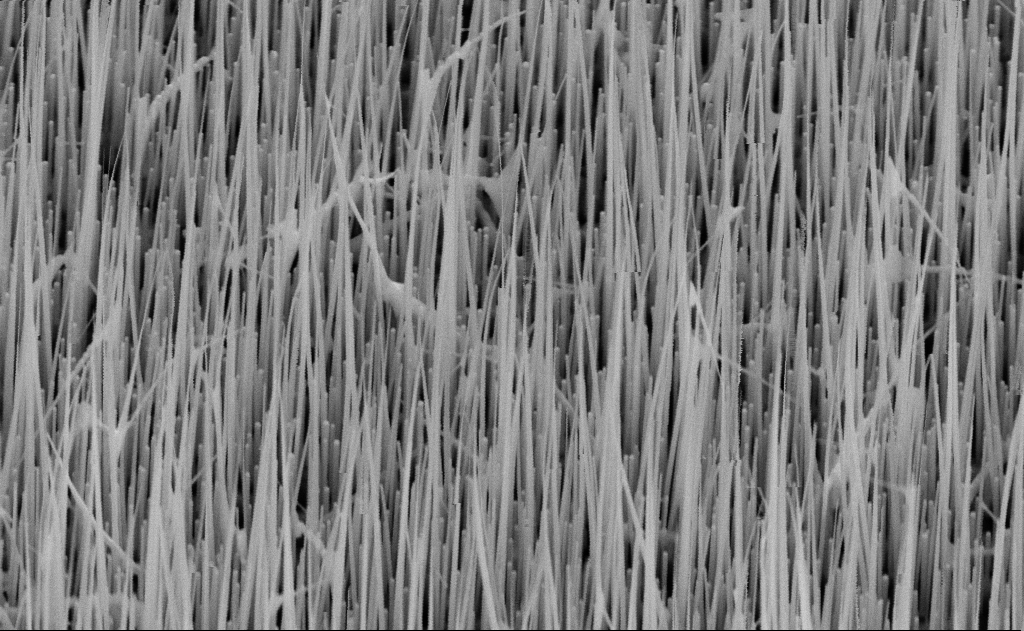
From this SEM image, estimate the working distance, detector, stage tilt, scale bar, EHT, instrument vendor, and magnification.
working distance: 16 mm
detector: SE2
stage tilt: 45°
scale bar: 1000 nm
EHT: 10 kV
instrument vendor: Zeiss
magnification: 40 K X